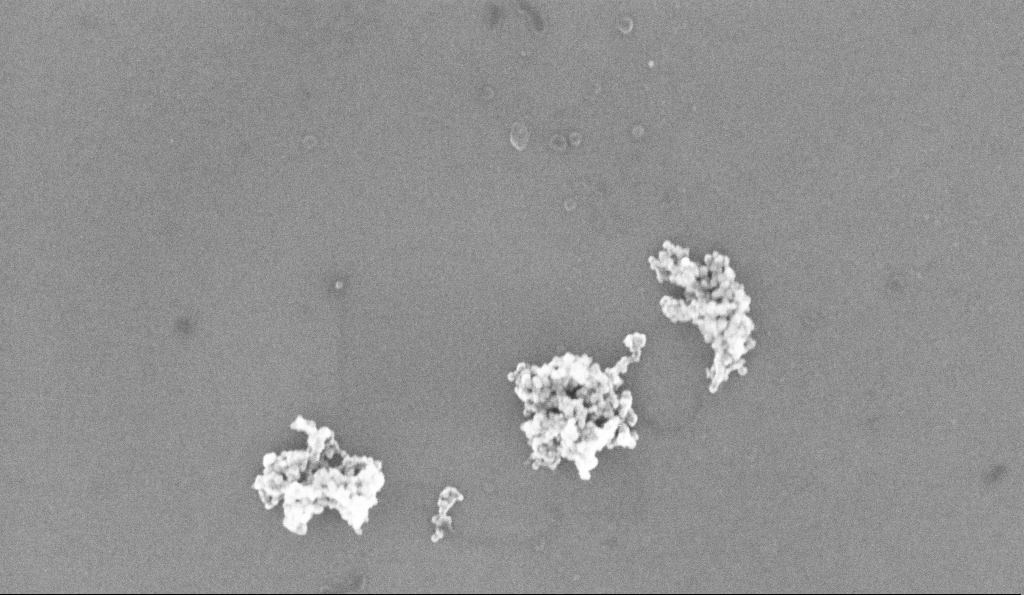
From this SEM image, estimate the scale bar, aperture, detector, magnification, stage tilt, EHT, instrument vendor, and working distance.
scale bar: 200 nm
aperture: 30 µm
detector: InLens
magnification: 152.82 K X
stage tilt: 0°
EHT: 10 kV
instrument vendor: Zeiss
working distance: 5.2 mm